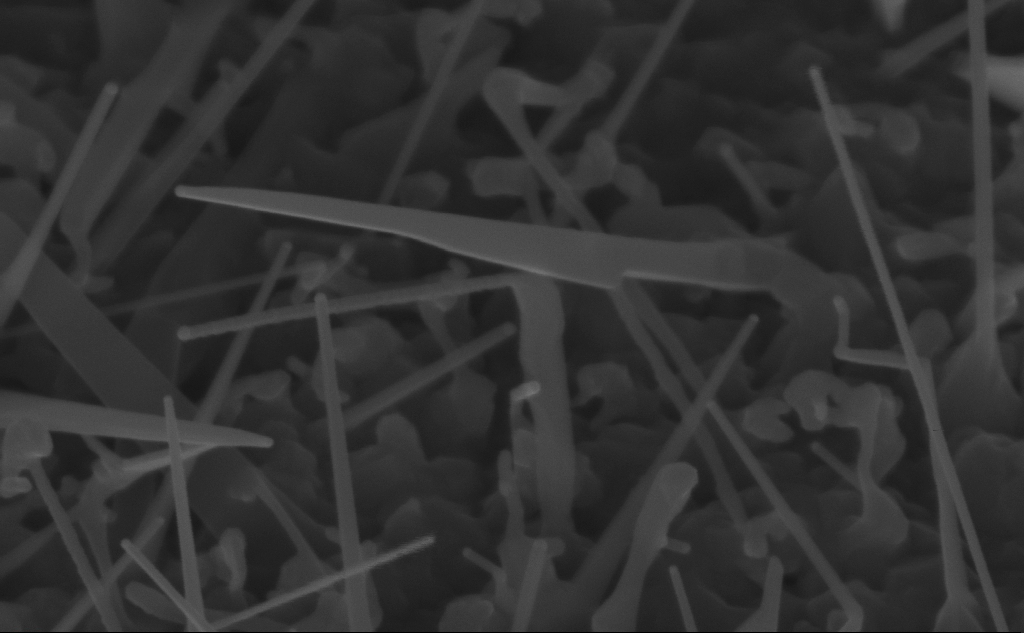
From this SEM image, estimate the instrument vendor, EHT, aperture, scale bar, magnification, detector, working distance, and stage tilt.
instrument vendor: Zeiss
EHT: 10 kV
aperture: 30 µm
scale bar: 200 nm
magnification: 150 K X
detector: InLens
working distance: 8 mm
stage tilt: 45°